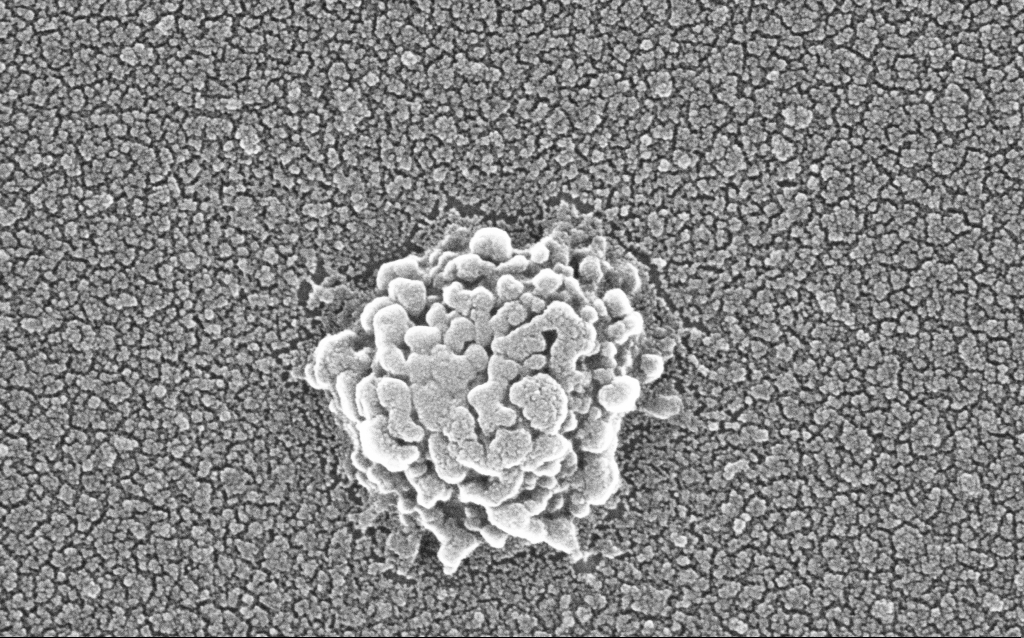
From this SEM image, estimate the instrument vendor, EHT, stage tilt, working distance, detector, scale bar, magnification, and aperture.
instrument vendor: Zeiss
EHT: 20 kV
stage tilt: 0°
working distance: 1.8 mm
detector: InLens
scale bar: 100 nm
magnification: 300 K X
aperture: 30 µm